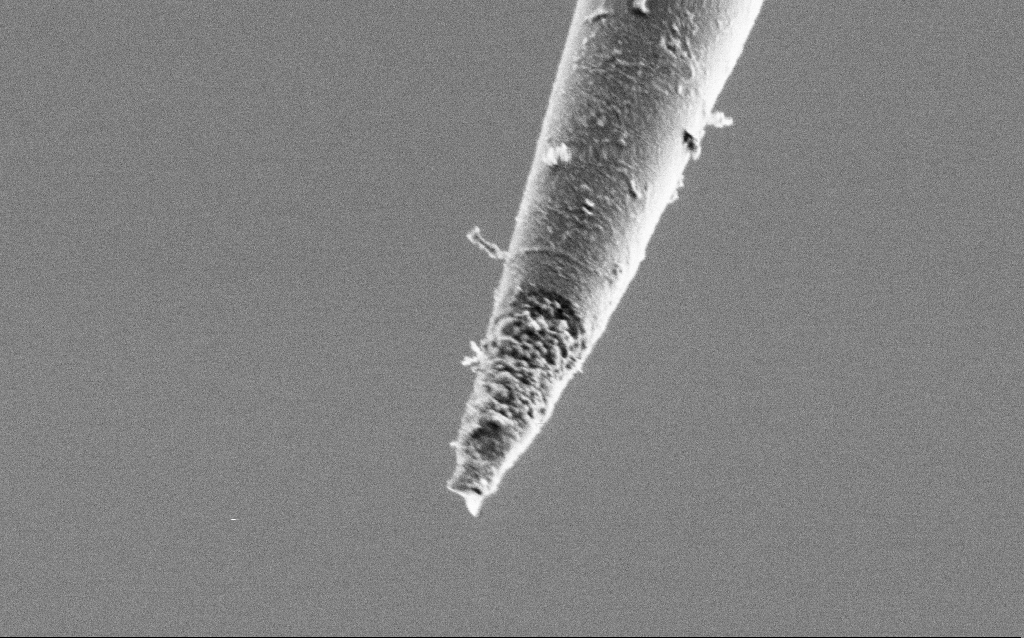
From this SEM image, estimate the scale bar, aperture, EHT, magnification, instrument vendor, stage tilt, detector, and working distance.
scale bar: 1000 nm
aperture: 30 µm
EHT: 1 kV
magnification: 50 K X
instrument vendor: Zeiss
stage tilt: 45°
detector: SE2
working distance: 6.5 mm